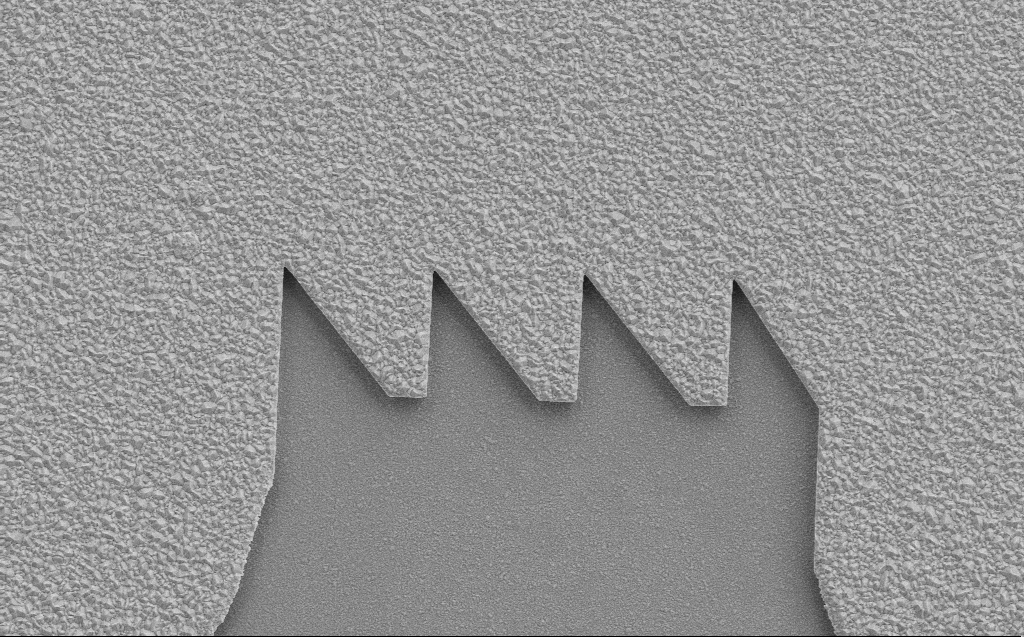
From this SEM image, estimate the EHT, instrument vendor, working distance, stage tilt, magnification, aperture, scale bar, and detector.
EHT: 10 kV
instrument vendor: Zeiss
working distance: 8 mm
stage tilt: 0°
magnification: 4.77 K X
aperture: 30 µm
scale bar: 10000 nm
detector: SE2